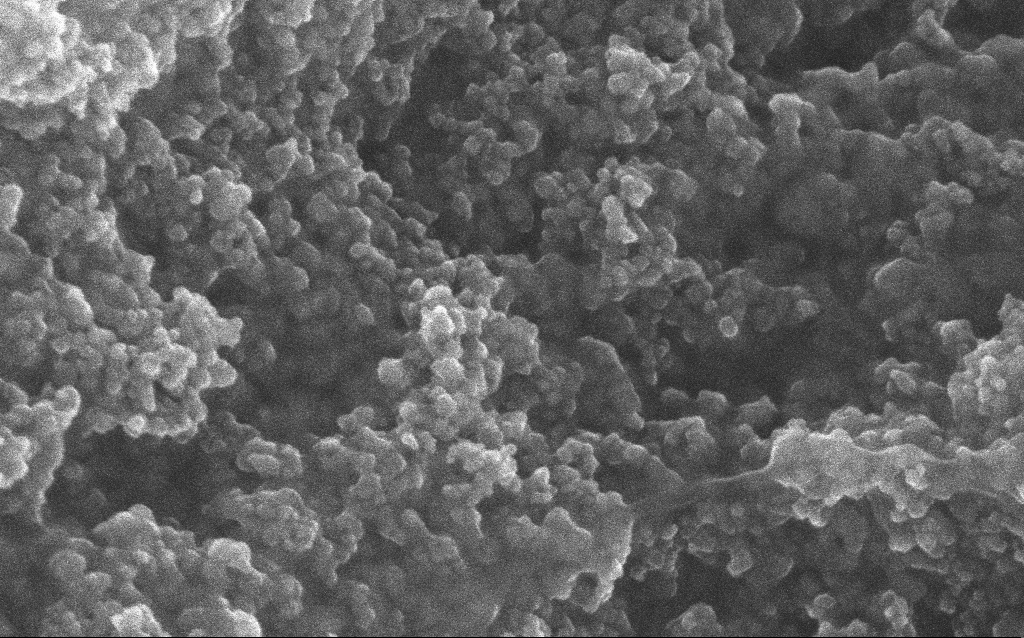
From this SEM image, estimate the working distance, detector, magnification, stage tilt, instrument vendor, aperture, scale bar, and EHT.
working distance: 2.8 mm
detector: InLens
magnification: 211.33 K X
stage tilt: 0°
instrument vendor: Zeiss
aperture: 30 µm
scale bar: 100 nm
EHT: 10 kV